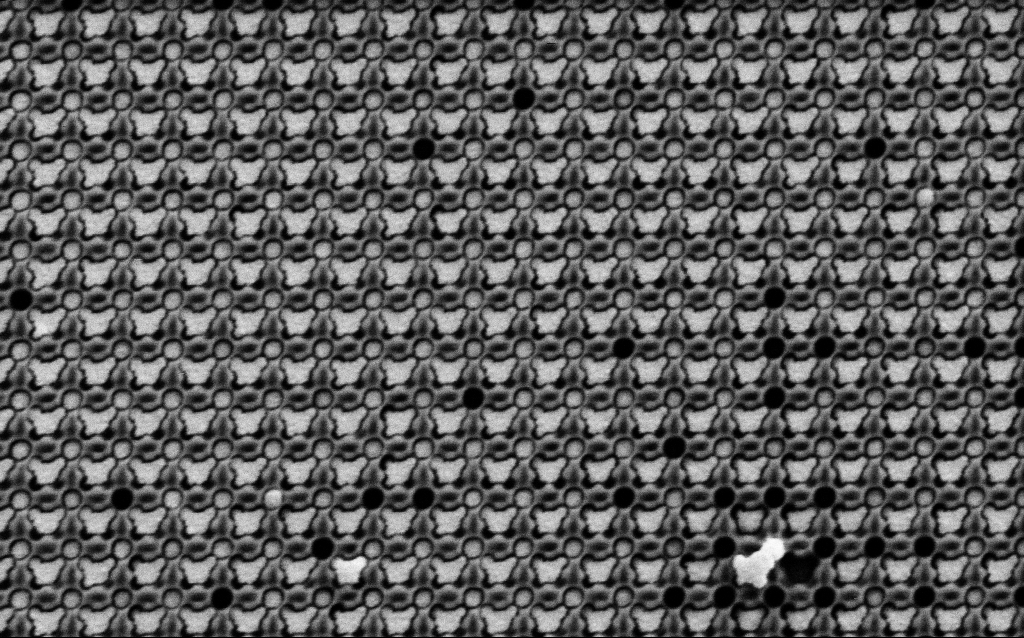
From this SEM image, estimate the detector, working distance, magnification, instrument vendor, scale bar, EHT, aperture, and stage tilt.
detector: SE2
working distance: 8 mm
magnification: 39.51 K X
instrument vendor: Zeiss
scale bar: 1000 nm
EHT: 1.5 kV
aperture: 30 µm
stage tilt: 0°